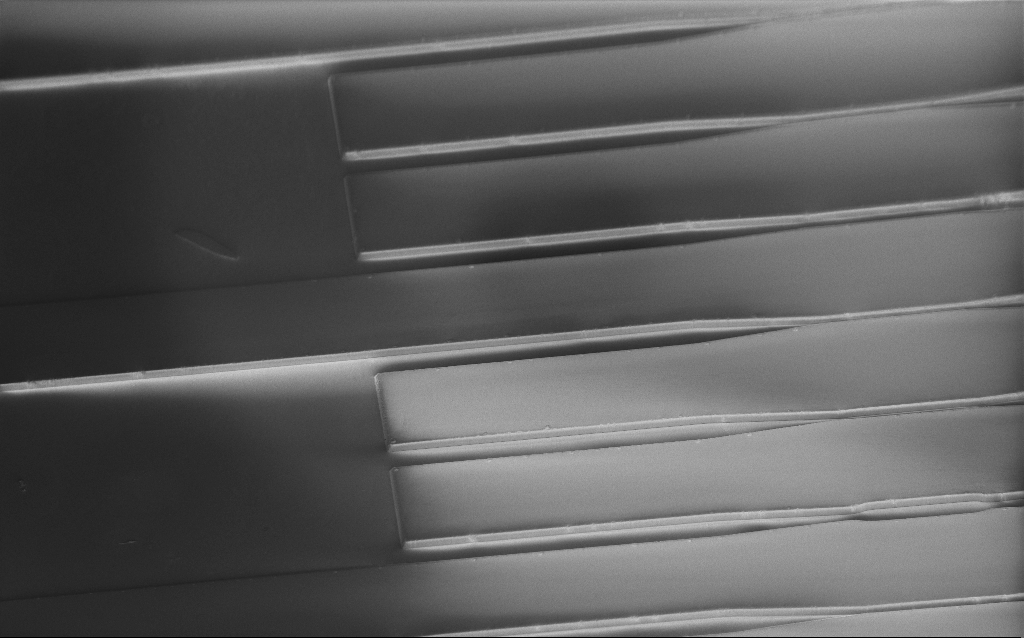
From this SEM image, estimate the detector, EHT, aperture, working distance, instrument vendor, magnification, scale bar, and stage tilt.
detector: InLens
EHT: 1.2 kV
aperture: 30 µm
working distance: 6 mm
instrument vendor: Zeiss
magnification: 0.484 K X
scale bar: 100000 nm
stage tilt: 45°